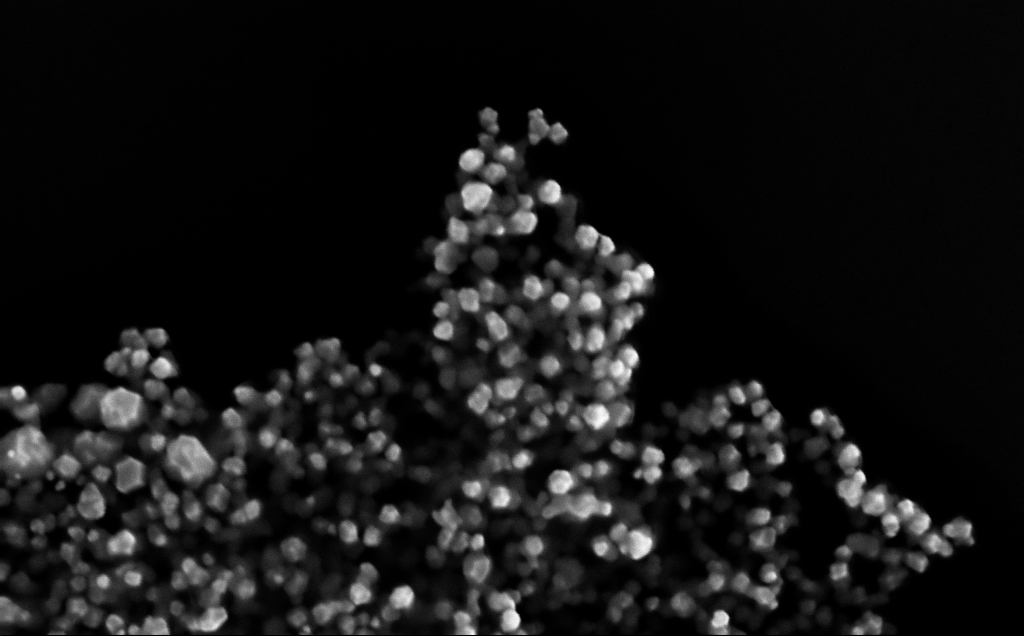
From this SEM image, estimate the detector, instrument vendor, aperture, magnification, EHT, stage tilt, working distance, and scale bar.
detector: InLens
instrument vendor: Zeiss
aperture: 30 µm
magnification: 186.06 K X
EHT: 10 kV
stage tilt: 0°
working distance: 4 mm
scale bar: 200 nm